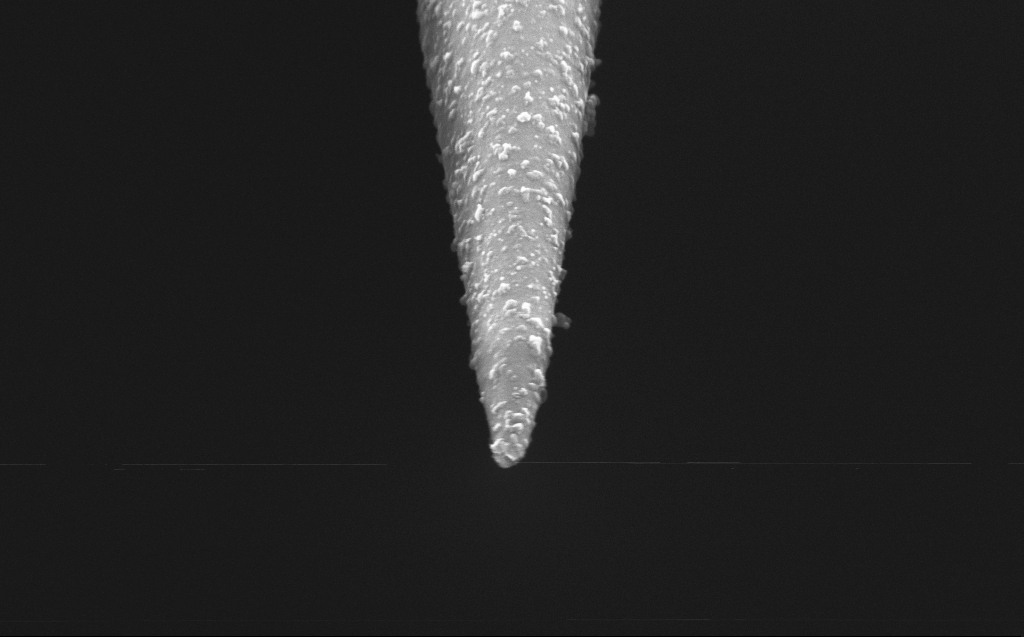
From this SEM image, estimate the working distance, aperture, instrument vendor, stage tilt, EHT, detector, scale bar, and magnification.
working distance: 3 mm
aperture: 30 µm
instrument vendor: Zeiss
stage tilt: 45°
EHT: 5 kV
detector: InLens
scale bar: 200 nm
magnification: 100 K X